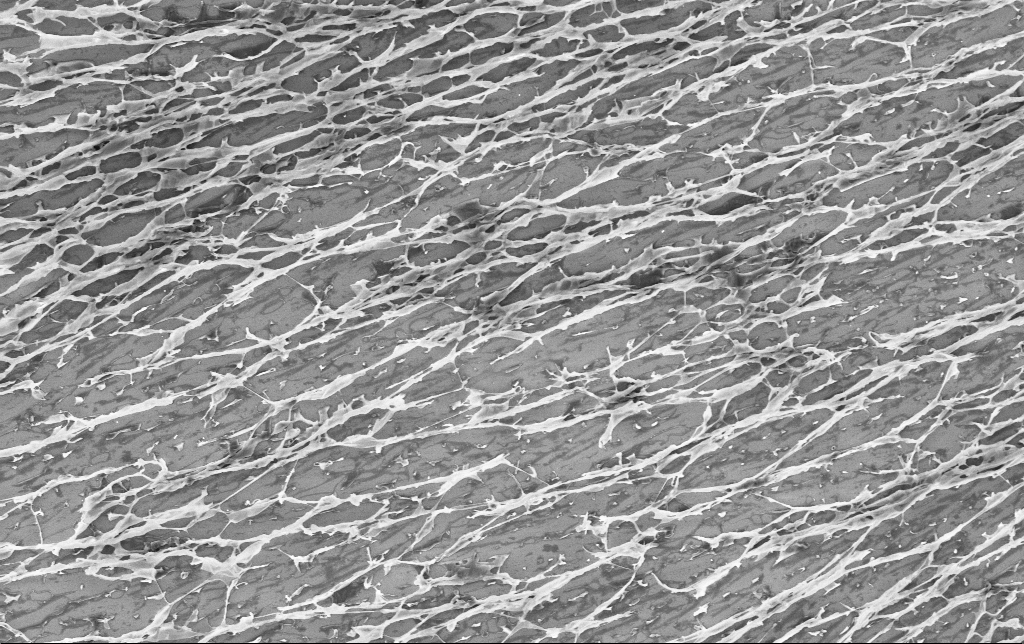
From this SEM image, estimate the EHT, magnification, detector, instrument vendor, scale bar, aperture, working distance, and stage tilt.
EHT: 3 kV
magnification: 7.35 K X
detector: InLens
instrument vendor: Zeiss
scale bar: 2000 nm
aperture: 30 µm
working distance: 3.4 mm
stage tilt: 0°